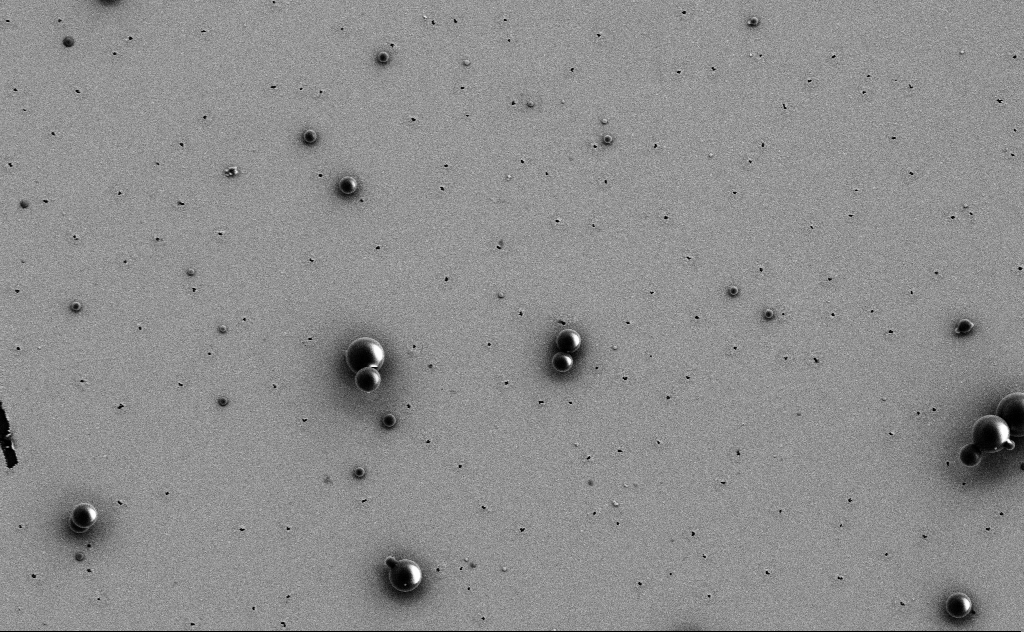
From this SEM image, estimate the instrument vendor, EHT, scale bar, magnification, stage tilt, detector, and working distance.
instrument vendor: Zeiss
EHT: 3 kV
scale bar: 2000 nm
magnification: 7.47 K X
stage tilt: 0°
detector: SE2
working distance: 10 mm